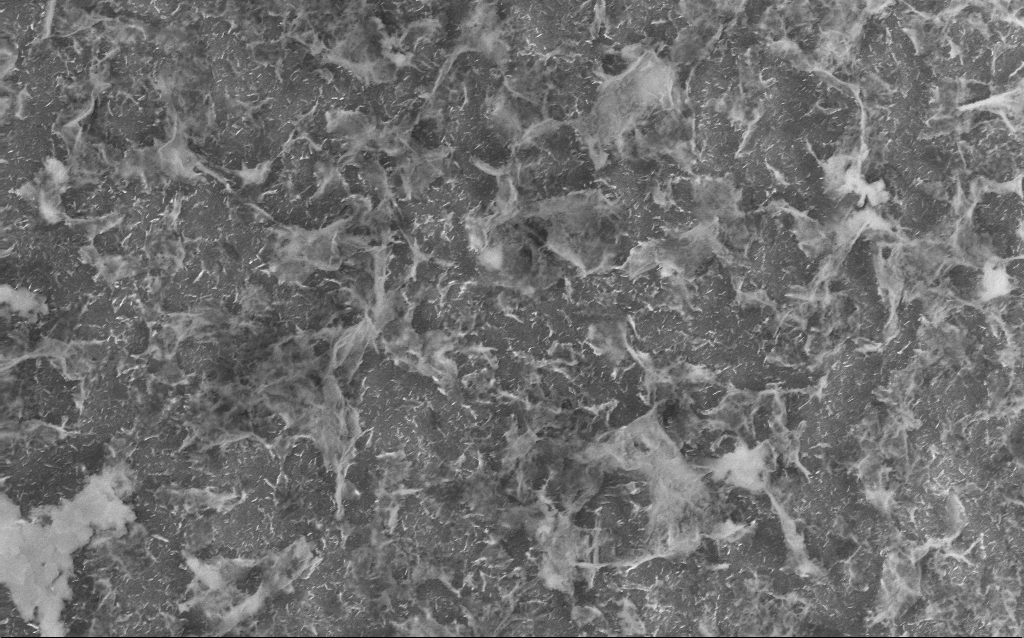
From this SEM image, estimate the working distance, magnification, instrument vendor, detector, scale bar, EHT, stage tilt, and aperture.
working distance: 2.5 mm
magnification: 20 K X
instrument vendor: Zeiss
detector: InLens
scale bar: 1000 nm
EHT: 10 kV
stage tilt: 0°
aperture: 30 µm